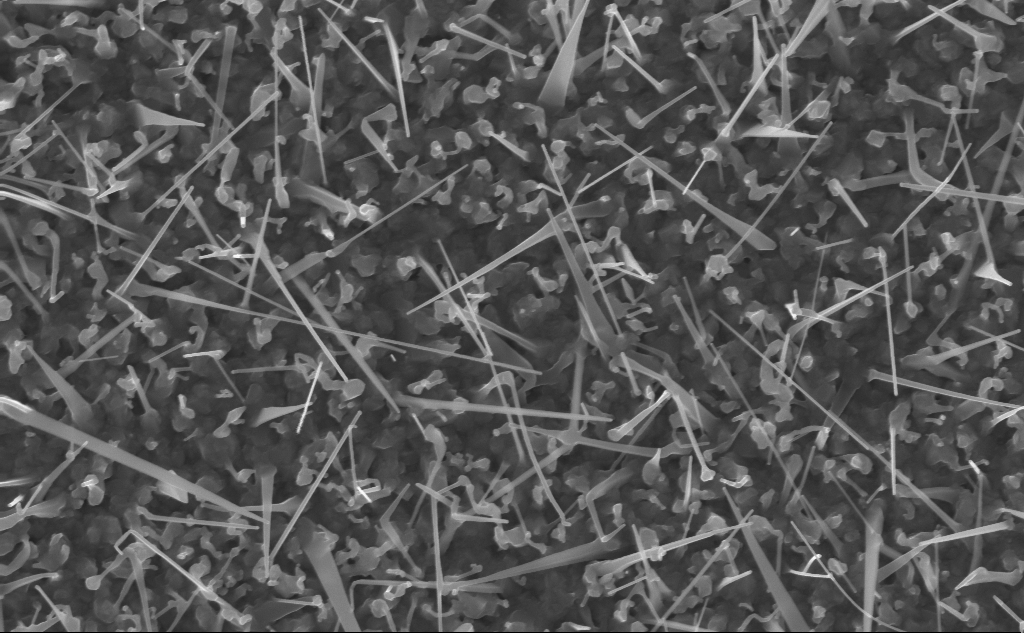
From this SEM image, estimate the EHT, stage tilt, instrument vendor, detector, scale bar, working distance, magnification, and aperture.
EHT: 10 kV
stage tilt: -0°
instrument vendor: Zeiss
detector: InLens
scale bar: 1000 nm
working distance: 5 mm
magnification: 40 K X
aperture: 30 µm